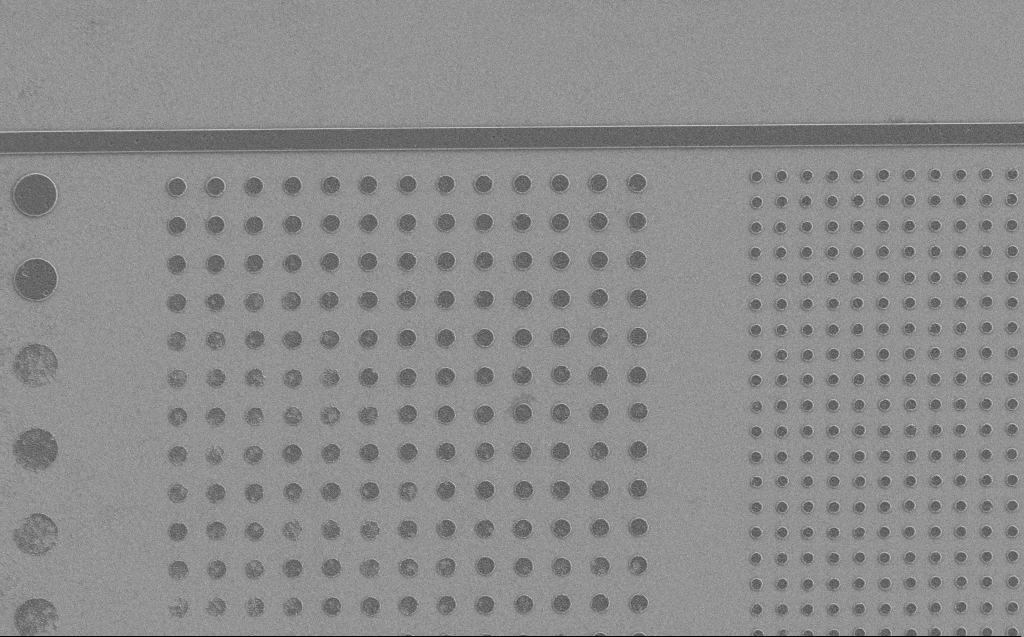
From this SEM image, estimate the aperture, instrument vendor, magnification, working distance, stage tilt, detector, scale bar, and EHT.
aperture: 30 µm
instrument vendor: Zeiss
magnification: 7.86 K X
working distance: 5 mm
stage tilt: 0°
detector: SE2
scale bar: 2000 nm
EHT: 1.2 kV